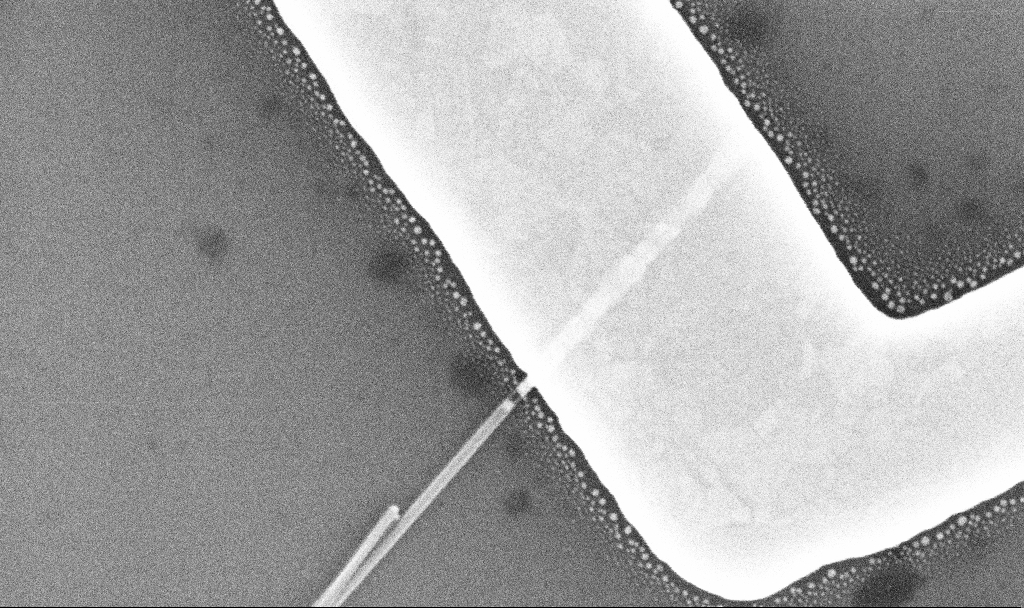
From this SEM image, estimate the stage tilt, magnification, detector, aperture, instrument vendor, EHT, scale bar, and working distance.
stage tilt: -0°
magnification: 148.88 K X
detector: InLens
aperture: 30 µm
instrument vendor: Zeiss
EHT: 10 kV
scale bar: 200 nm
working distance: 7 mm